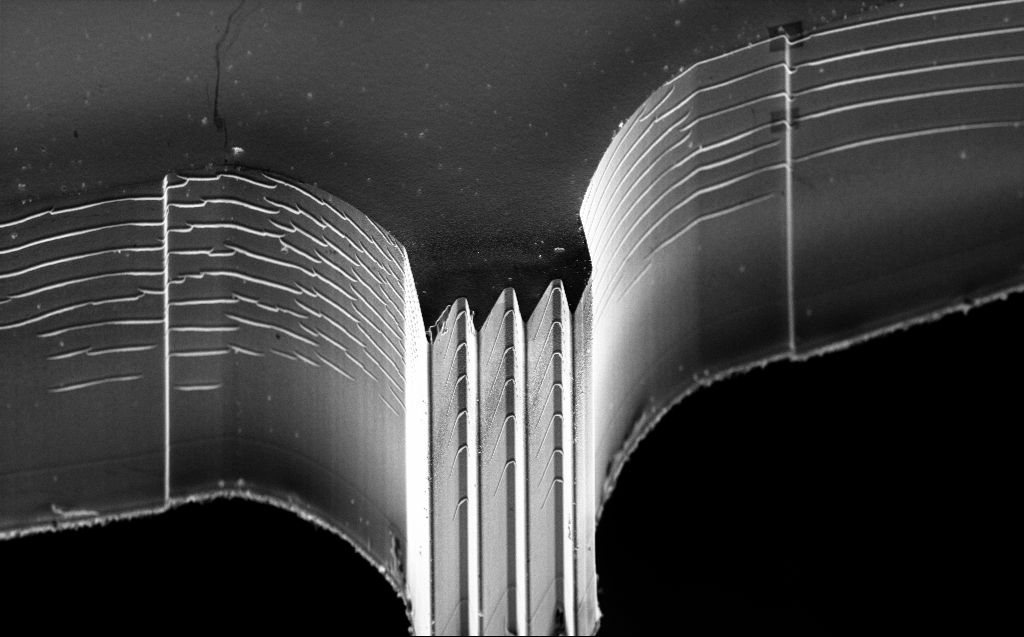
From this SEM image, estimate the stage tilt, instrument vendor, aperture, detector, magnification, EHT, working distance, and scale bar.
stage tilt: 45°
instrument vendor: Zeiss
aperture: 30 µm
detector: InLens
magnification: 1.59 K X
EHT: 10 kV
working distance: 4 mm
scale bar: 10000 nm